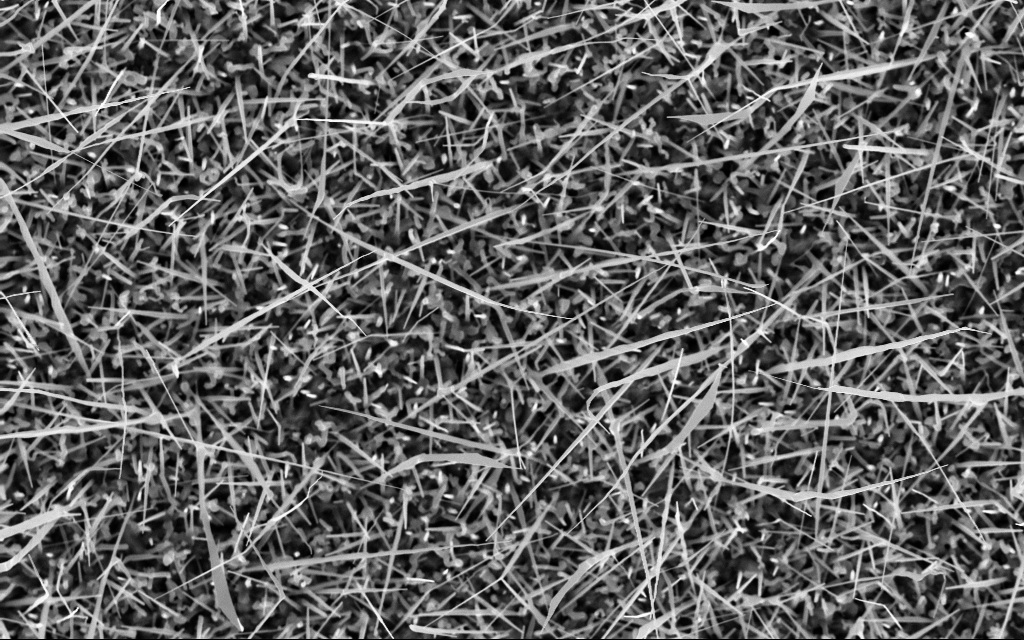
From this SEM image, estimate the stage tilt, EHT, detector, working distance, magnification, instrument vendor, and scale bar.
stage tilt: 0°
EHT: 10 kV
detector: InLens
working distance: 6 mm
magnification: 20 K X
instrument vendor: Zeiss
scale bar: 2000 nm